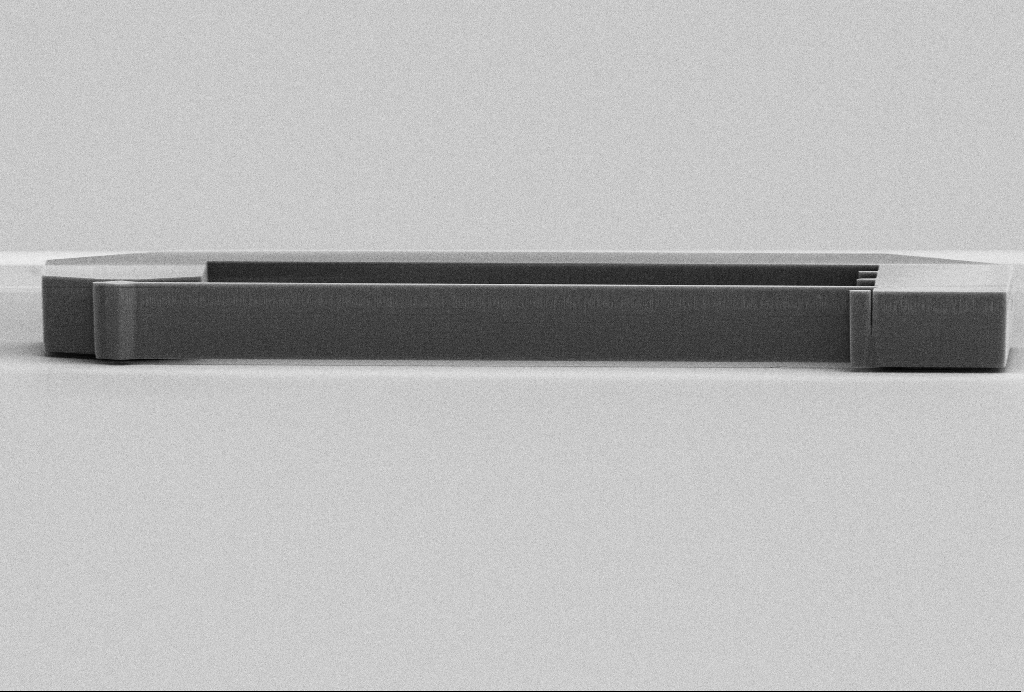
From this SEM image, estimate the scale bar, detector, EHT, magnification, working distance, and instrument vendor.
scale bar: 20000 nm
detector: InLens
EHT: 10 kV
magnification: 1.93 K X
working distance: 18.8 mm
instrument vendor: Zeiss